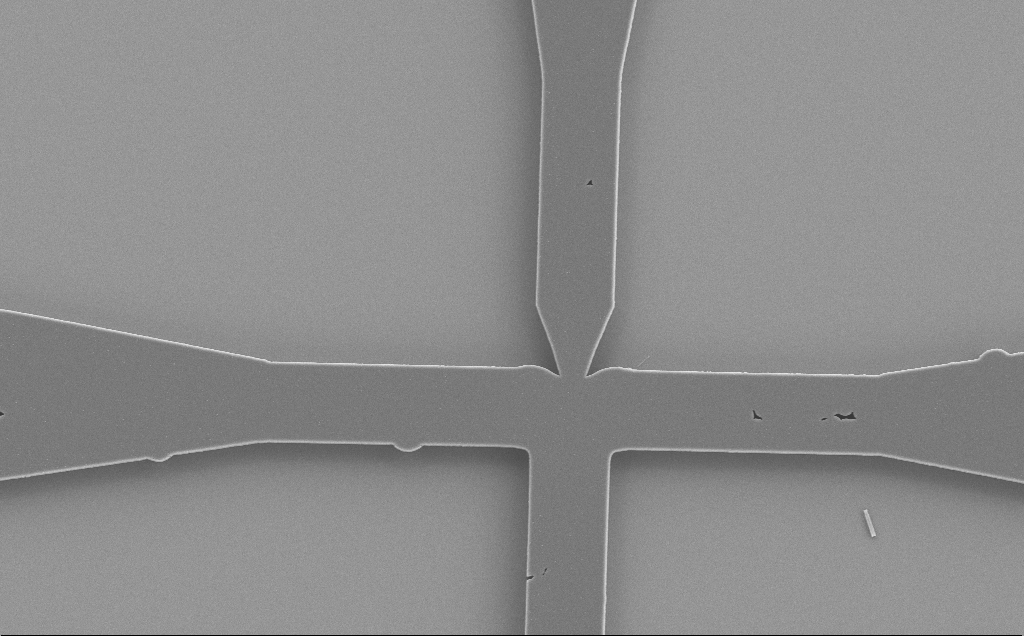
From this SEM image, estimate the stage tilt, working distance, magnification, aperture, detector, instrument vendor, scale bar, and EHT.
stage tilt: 0°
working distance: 9 mm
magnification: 2.86 K X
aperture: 30 µm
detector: SE2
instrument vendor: Zeiss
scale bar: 10000 nm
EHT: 5 kV